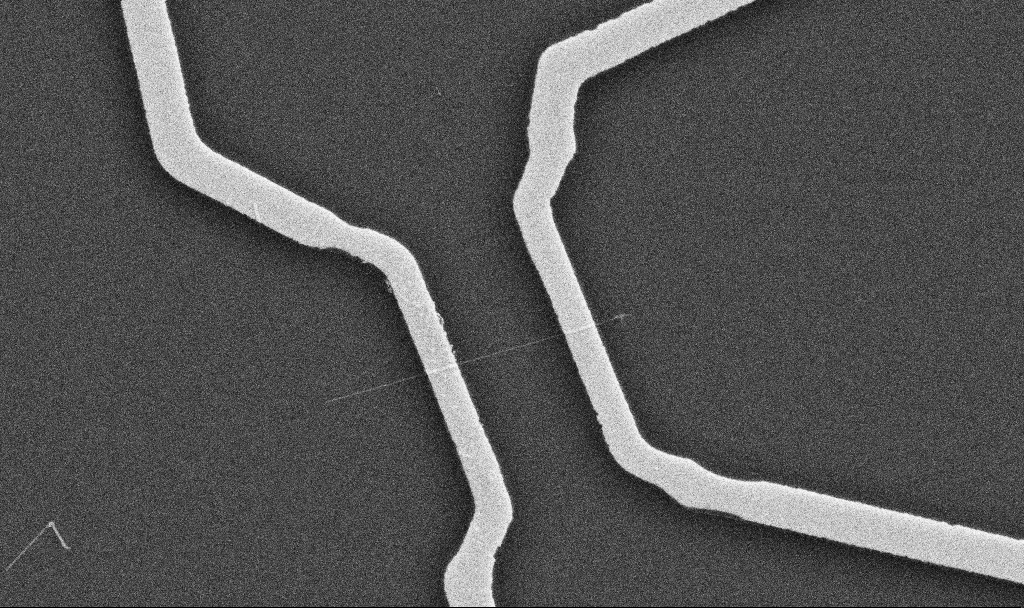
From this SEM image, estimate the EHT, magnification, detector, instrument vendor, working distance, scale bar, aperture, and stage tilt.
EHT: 10 kV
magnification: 20 K X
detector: SE2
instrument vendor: Zeiss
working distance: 10.7 mm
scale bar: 1000 nm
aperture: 30 µm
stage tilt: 0°